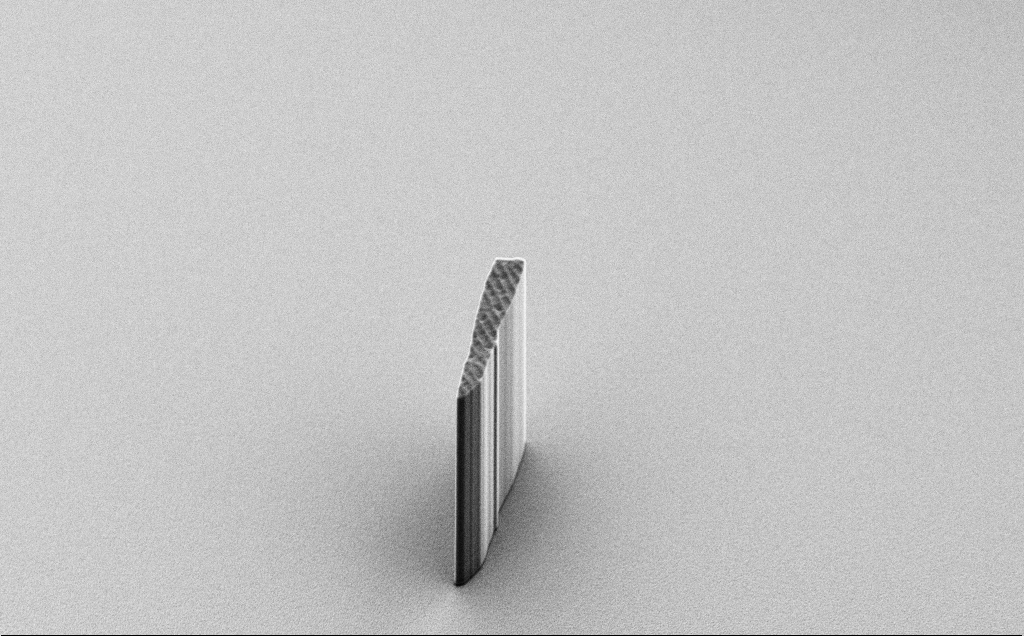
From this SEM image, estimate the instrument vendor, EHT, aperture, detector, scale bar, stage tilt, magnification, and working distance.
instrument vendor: Zeiss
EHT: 10 kV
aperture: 30 µm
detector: SE2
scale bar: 100000 nm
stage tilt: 45°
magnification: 0.665 K X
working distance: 8 mm